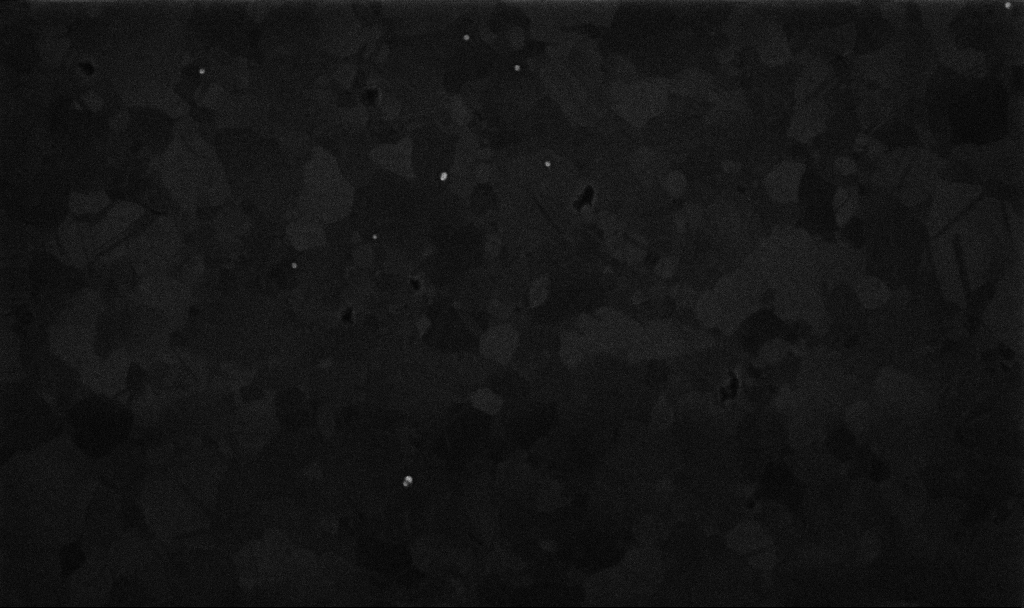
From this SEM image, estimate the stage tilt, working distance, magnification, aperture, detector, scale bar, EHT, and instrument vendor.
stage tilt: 0°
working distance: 3.3 mm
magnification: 100 K X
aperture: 30 µm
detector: InLens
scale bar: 200 nm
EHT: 10 kV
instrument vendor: Zeiss